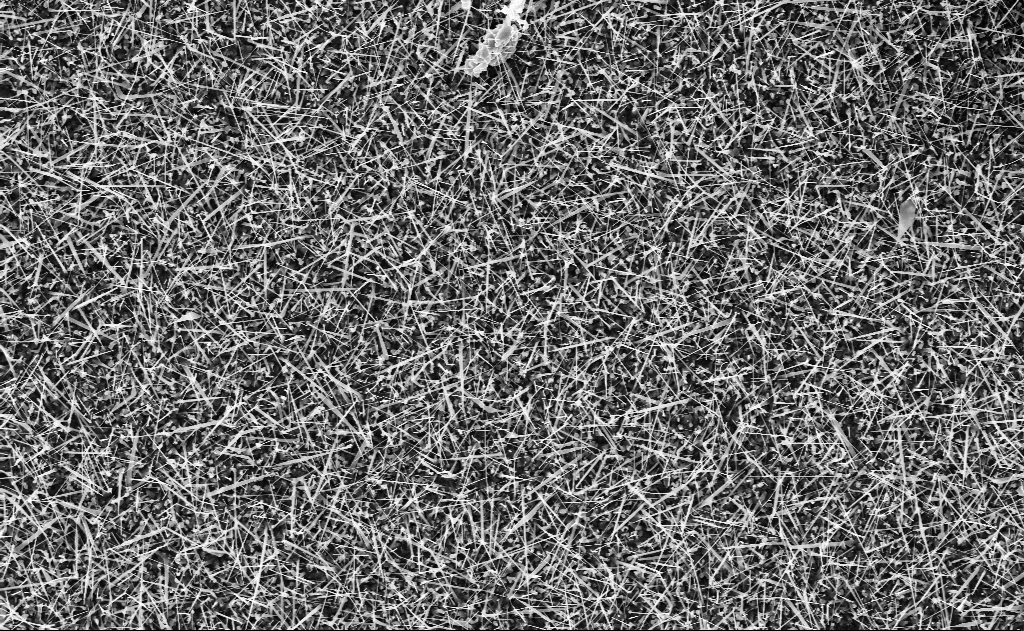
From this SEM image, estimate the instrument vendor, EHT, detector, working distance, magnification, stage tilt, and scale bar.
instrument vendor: Zeiss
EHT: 10 kV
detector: InLens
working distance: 12 mm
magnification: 10 K X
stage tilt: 0°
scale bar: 2000 nm